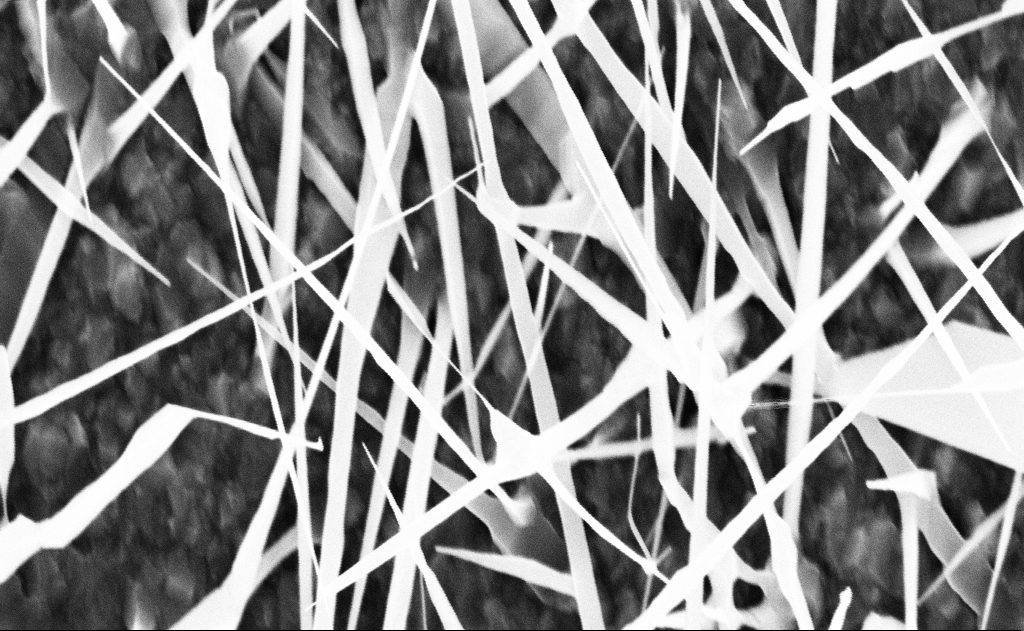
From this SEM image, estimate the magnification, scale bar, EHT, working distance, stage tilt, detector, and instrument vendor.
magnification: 40 K X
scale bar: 1000 nm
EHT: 10 kV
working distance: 16 mm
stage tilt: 0°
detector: InLens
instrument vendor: Zeiss